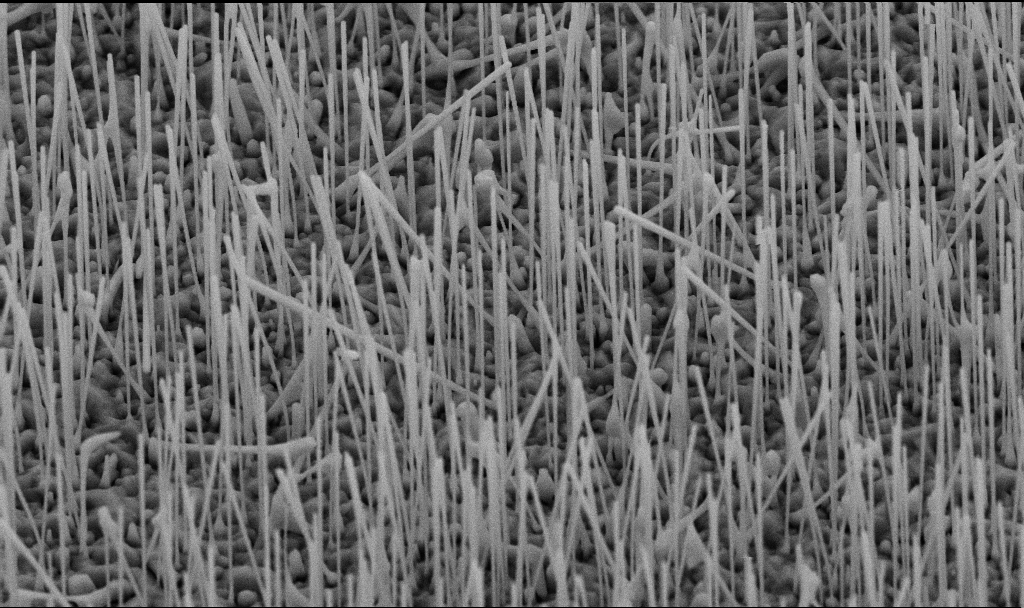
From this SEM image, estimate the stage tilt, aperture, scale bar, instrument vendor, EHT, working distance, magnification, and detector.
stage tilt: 45°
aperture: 30 µm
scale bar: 2000 nm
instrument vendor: Zeiss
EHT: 10 kV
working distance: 7.2 mm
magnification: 15 K X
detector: SE2